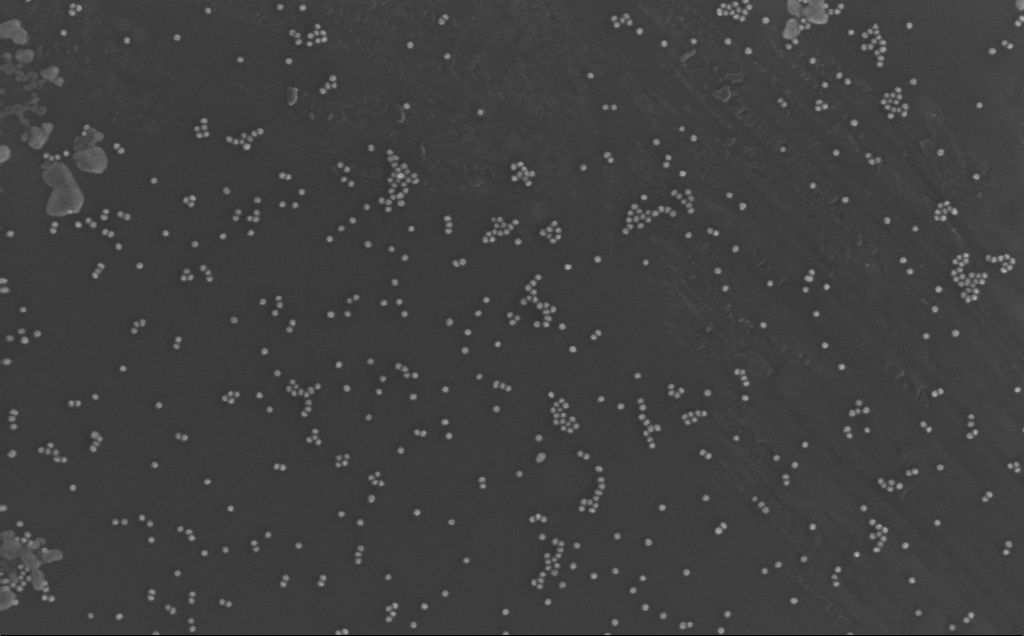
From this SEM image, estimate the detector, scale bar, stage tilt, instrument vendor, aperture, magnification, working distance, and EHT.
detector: InLens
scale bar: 200 nm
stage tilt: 0°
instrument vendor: Zeiss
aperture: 30 µm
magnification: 131.29 K X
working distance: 3 mm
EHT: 10 kV